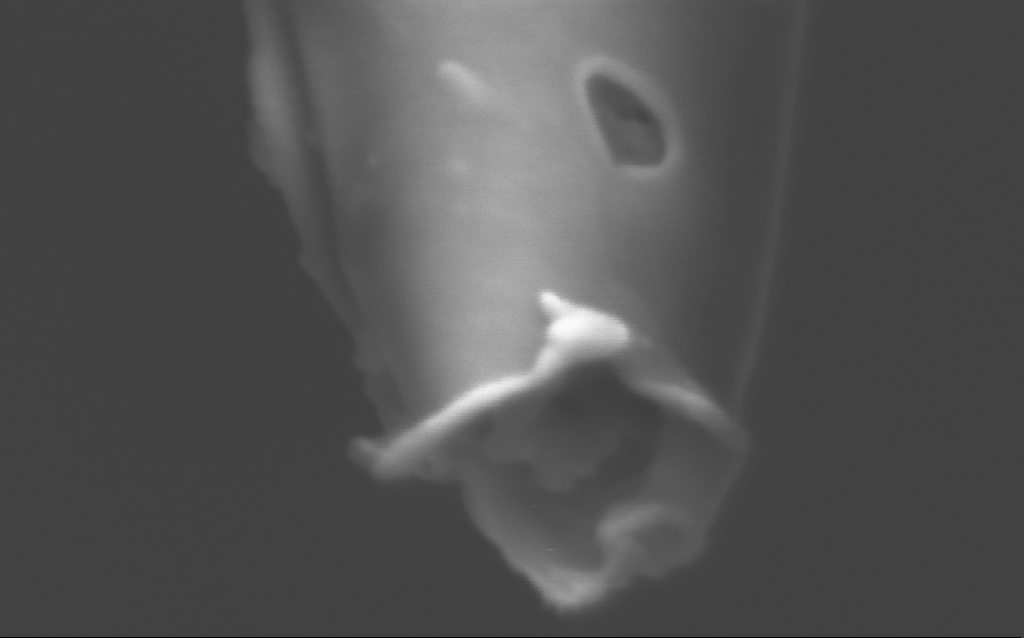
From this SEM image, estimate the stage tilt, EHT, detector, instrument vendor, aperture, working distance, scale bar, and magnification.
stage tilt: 45°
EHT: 2 kV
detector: InLens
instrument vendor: Zeiss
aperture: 30 µm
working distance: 6 mm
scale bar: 100 nm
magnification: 500 K X